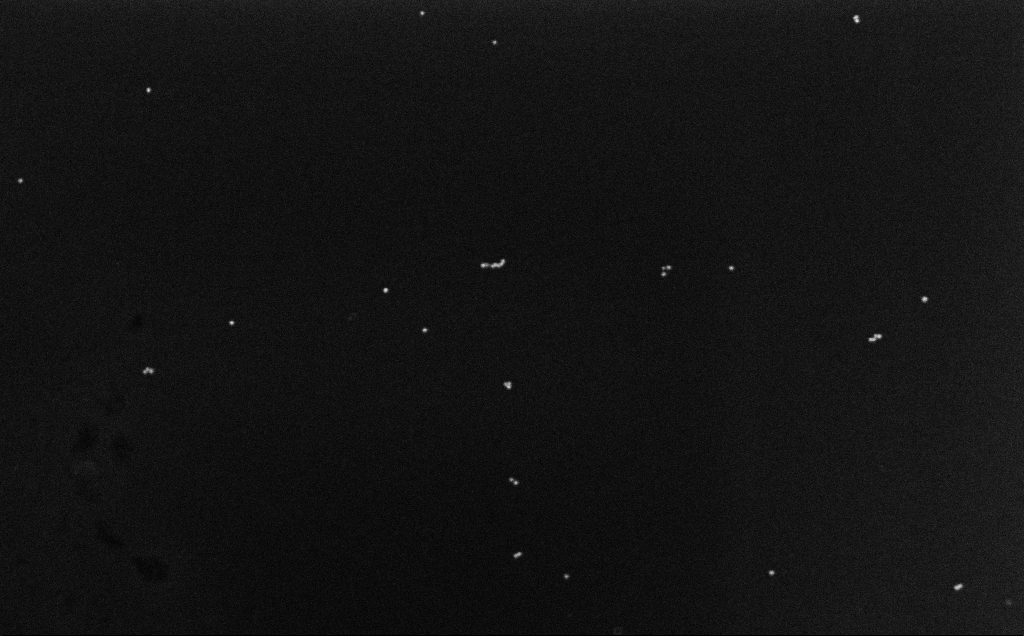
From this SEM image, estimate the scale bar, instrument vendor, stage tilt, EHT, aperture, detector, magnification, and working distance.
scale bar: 200 nm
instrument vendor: Zeiss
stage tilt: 0°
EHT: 10 kV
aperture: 30 µm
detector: InLens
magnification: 100 K X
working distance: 6.6 mm